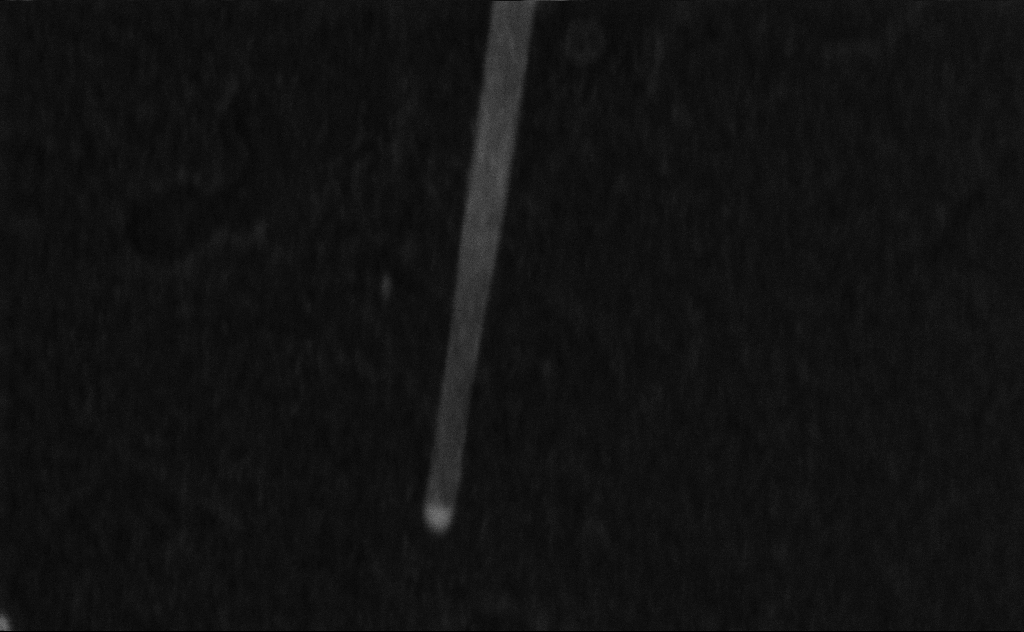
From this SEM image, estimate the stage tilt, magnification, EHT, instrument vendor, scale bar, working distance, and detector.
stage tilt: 0°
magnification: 511.45 K X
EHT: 20 kV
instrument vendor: Zeiss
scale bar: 100 nm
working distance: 8 mm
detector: InLens